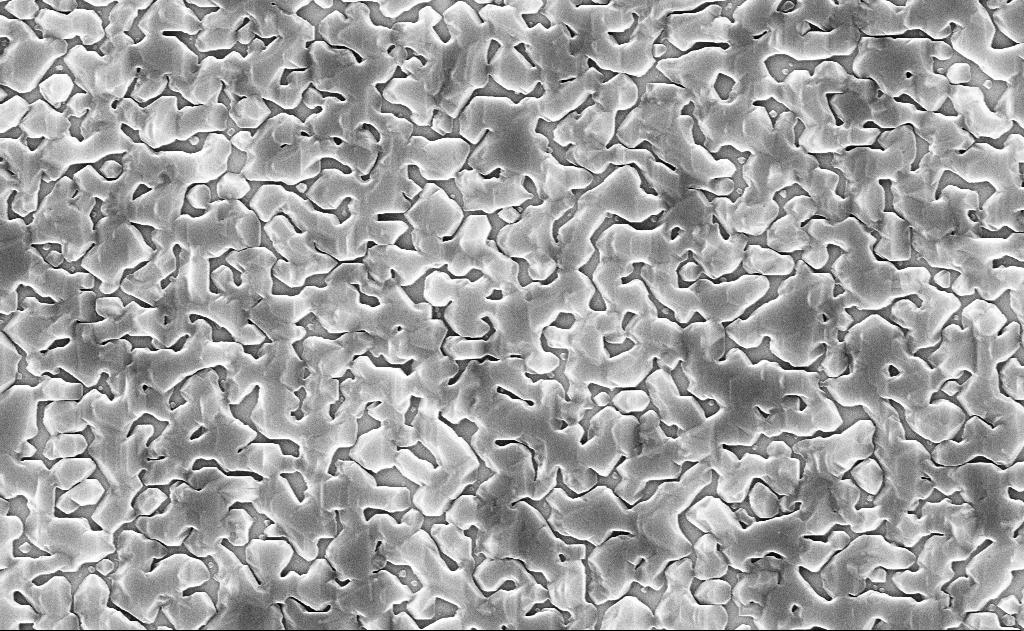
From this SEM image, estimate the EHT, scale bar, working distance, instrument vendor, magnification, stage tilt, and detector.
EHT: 10 kV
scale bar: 1000 nm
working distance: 11 mm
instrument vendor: Zeiss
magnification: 50 K X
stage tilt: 0°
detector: InLens